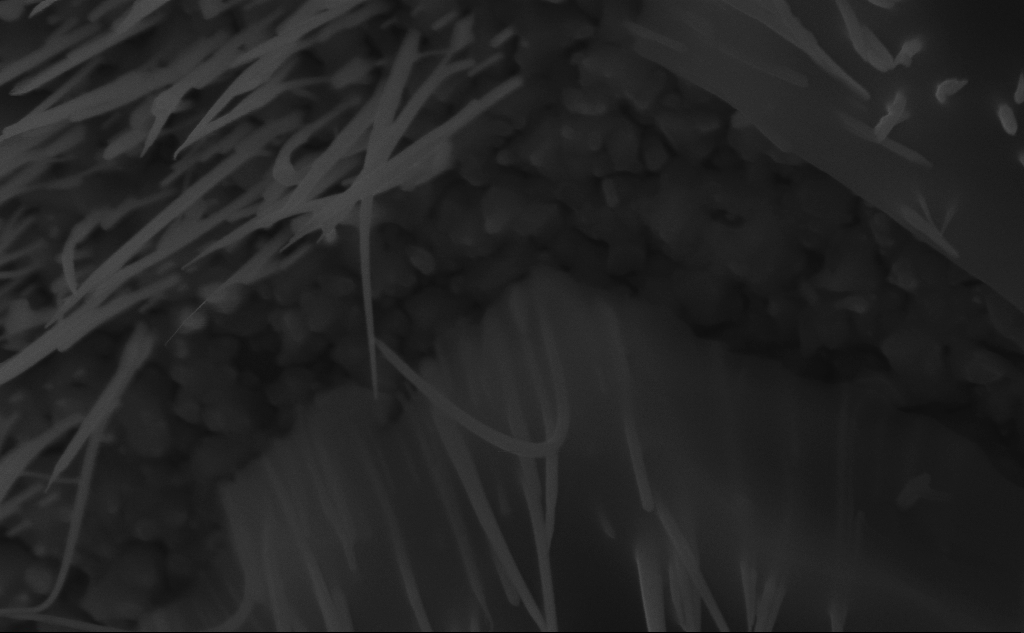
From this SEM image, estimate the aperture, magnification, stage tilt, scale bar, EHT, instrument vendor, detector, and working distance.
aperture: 30 µm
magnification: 98.96 K X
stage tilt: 45°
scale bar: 200 nm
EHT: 10 kV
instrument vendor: Zeiss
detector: InLens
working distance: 6 mm